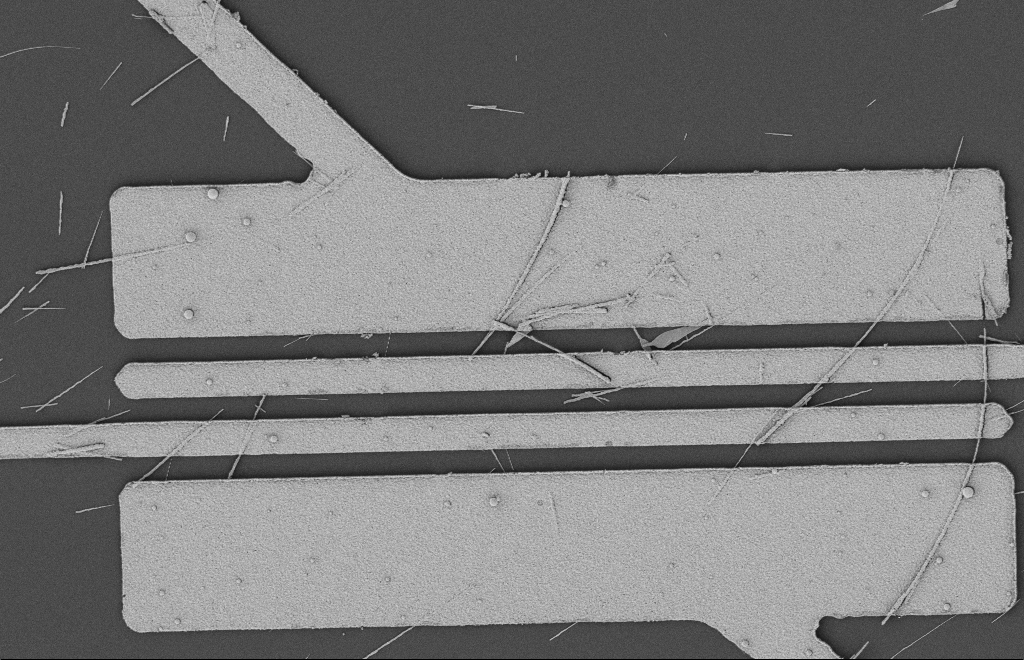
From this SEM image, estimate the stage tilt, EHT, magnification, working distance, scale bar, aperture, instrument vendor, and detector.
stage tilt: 0°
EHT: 2 kV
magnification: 5.37 K X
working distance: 12 mm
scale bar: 2000 nm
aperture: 20 µm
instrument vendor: Zeiss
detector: SE2